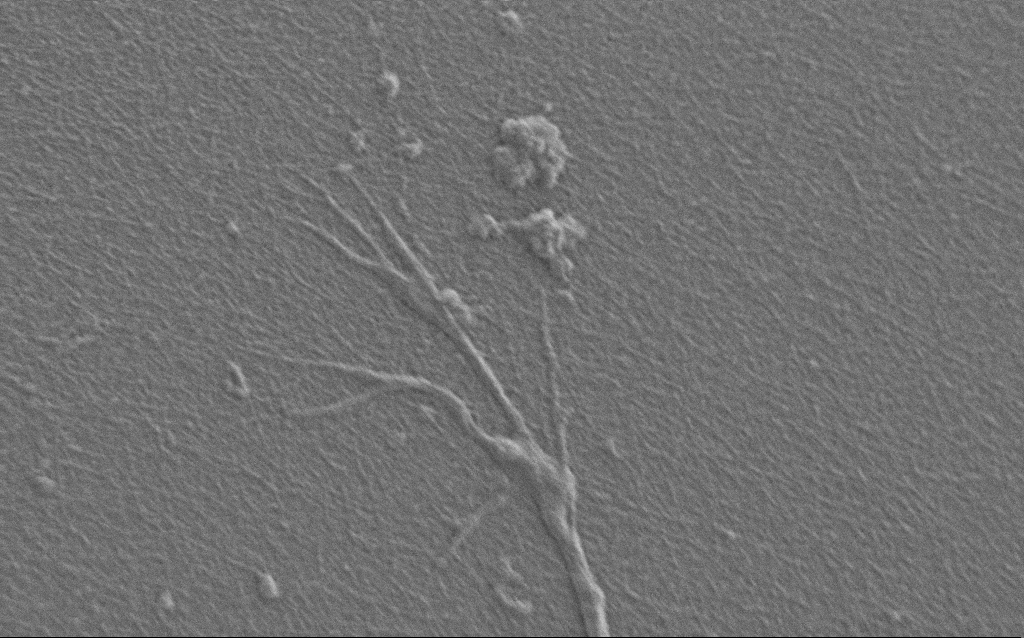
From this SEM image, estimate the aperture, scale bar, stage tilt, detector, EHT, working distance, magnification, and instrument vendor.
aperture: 30 µm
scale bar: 2000 nm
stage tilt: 0°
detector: SE2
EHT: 0.9 kV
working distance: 7 mm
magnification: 10 K X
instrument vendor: Zeiss